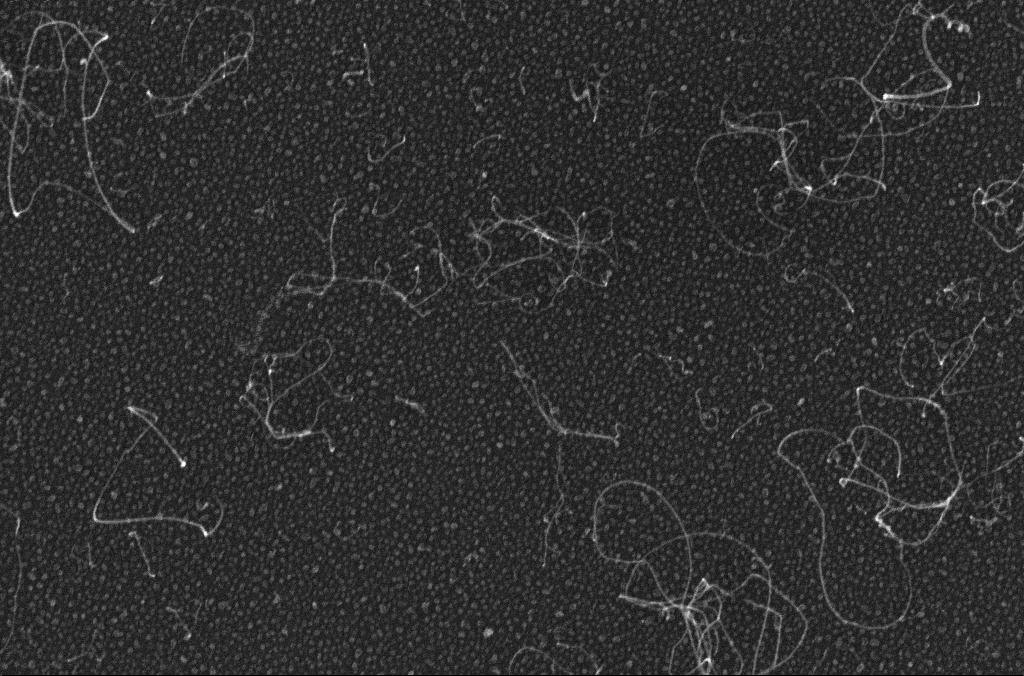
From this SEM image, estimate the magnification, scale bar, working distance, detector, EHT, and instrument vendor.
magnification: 100 K X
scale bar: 200 nm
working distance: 3.1 mm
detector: InLens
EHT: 10 kV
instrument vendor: Zeiss